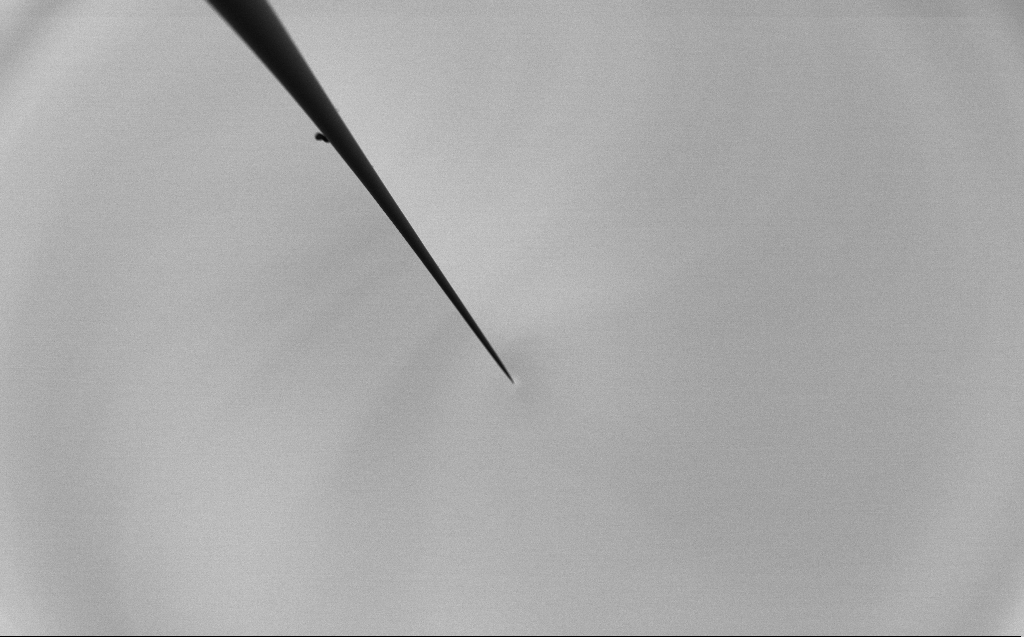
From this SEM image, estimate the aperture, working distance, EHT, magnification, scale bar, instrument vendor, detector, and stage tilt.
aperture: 30 µm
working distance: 4 mm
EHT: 2 kV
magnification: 0.1 K X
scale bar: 200000 nm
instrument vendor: Zeiss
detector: SE2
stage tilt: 45°